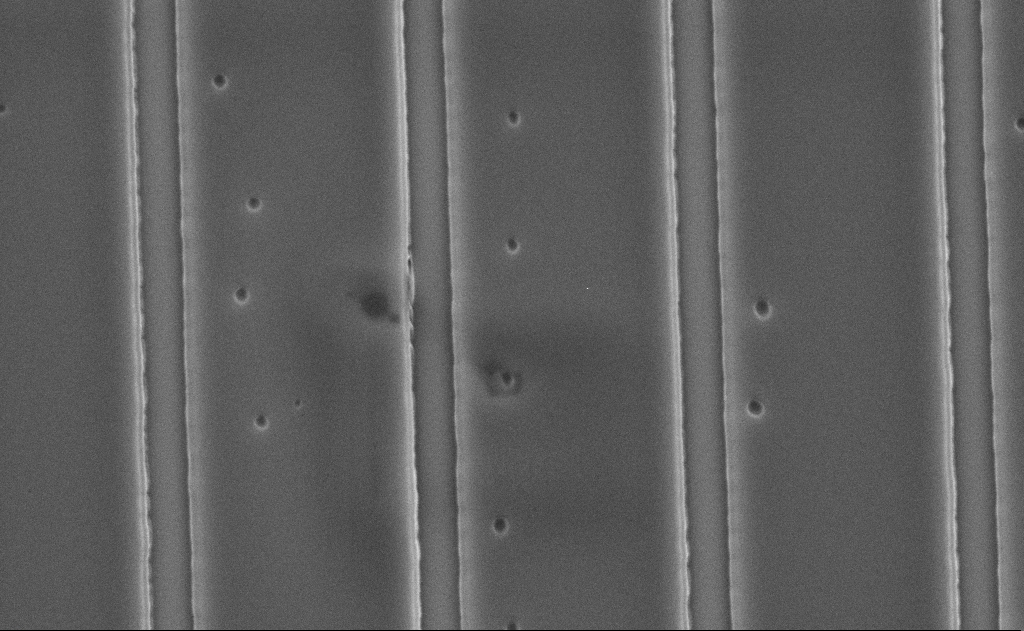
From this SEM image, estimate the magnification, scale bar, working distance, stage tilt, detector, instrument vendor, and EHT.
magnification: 24.43 K X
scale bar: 1000 nm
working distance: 15 mm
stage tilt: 0°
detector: SE2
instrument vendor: Zeiss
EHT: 5 kV